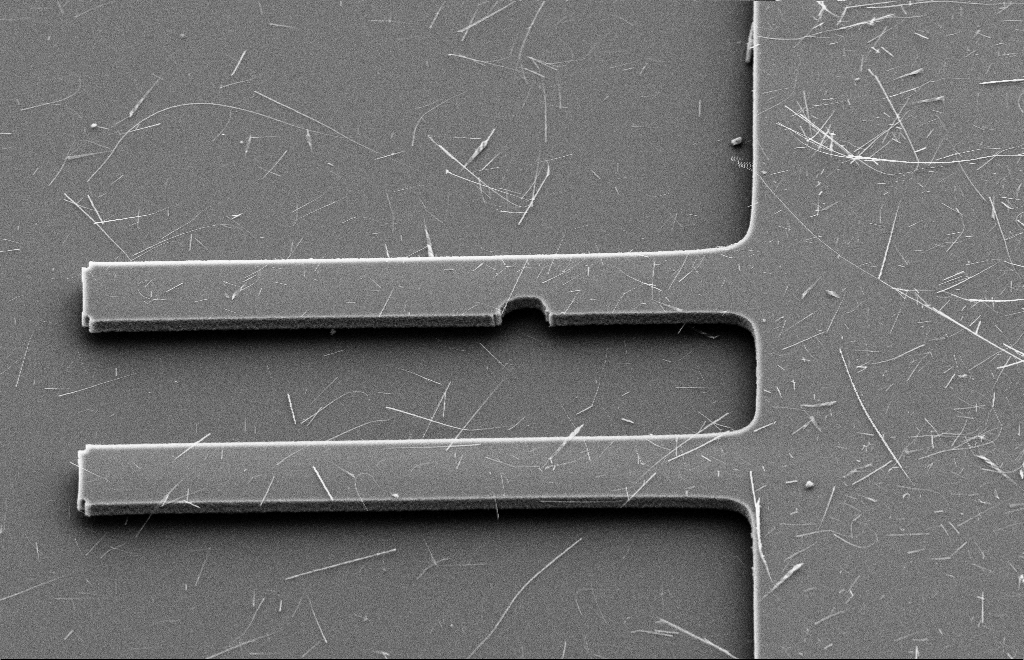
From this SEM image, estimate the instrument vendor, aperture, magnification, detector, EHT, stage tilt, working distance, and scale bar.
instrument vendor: Zeiss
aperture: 20 µm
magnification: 2.5 K X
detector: SE2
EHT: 10 kV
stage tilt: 39.8°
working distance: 9 mm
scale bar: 10000 nm